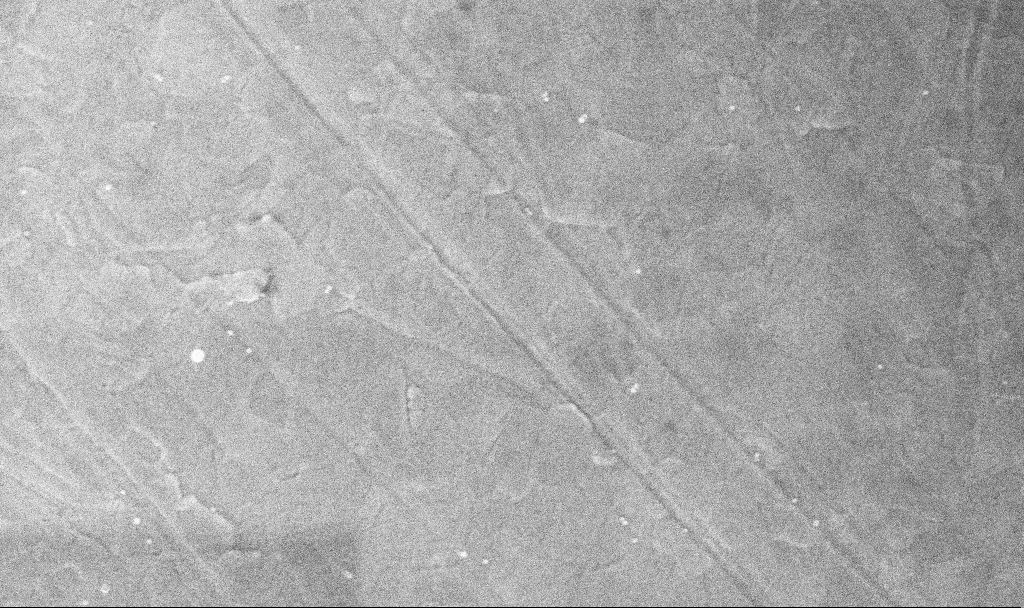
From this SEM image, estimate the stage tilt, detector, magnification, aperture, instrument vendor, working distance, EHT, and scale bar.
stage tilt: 0°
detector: InLens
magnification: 100 K X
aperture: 30 µm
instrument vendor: Zeiss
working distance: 3.7 mm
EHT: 5 kV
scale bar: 200 nm